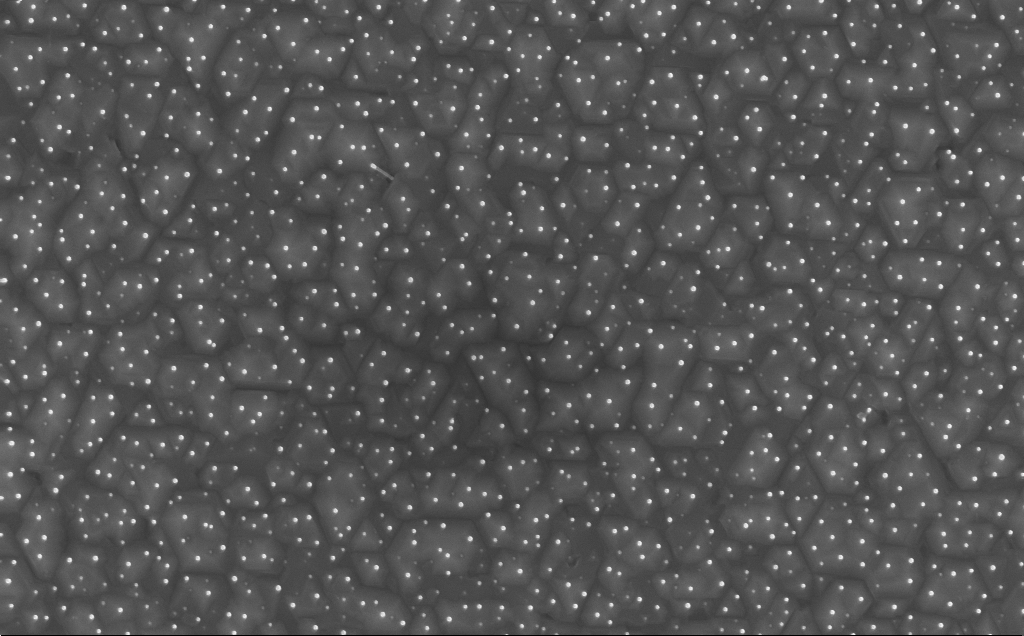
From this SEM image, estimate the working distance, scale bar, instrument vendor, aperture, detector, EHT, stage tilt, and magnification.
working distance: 6 mm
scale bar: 1000 nm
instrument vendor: Zeiss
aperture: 30 µm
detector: InLens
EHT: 10 kV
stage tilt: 0°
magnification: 40 K X